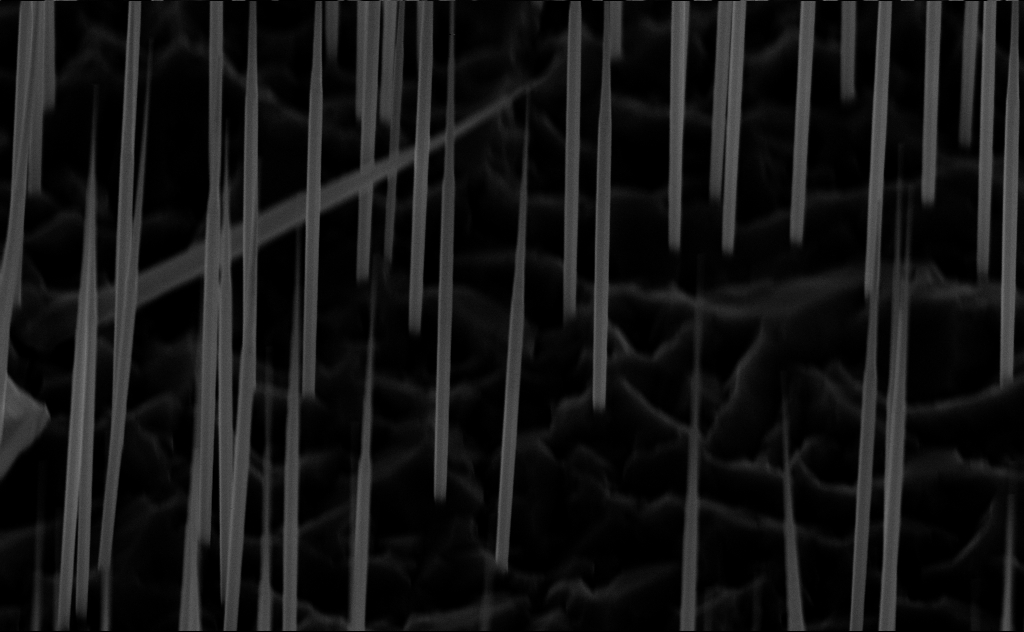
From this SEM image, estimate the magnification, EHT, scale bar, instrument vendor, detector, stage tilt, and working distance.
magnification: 34.46 K X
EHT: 10 kV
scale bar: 2000 nm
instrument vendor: Zeiss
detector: InLens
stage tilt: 45°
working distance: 7 mm